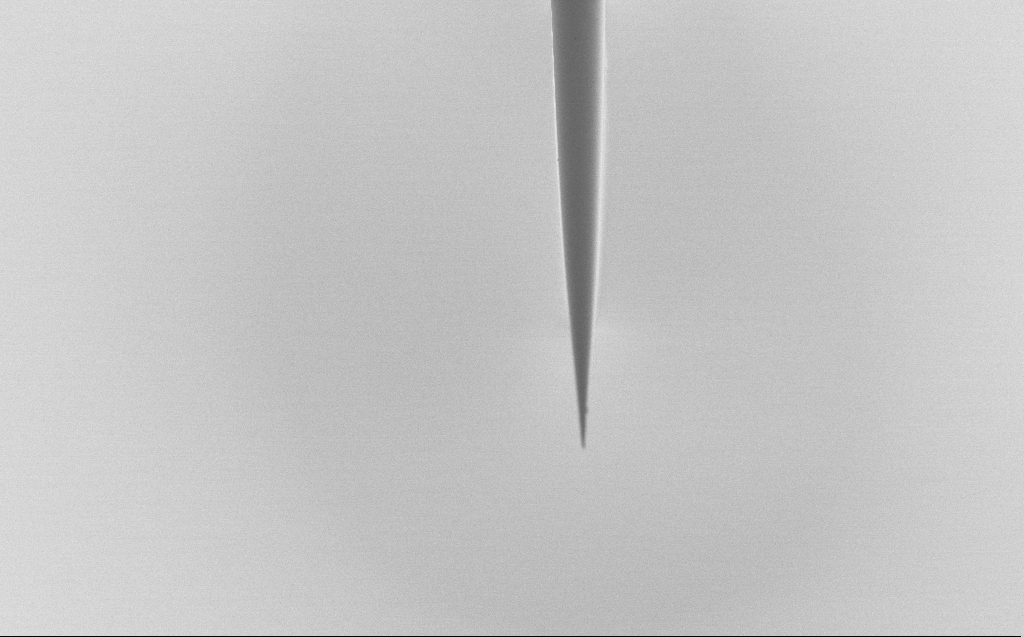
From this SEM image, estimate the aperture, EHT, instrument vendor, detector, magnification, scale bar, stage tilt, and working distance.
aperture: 30 µm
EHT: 1 kV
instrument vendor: Zeiss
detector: SE2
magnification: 1 K X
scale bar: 20000 nm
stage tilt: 45°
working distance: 5 mm